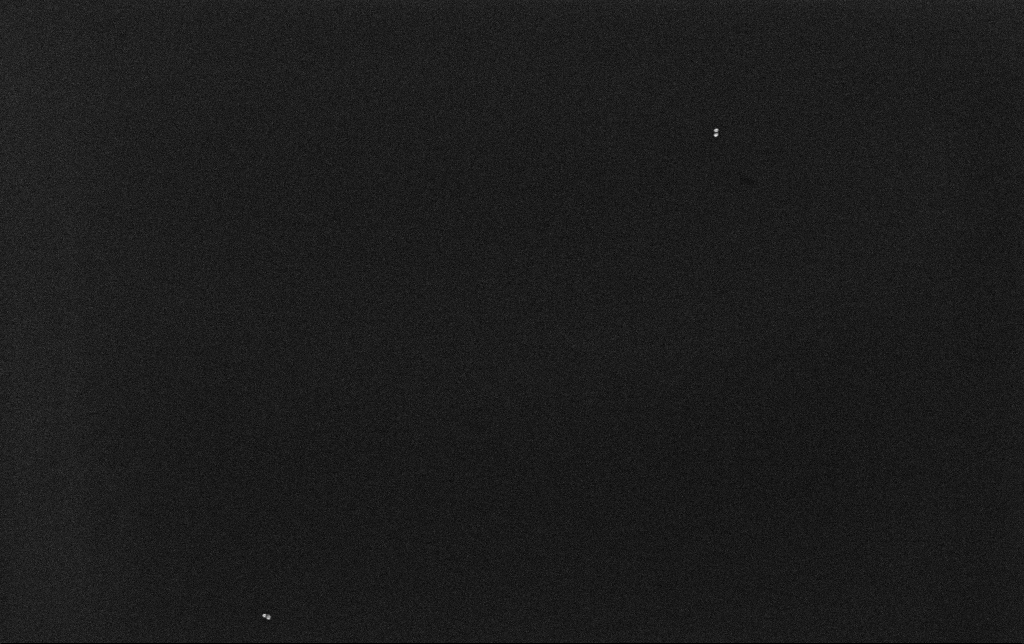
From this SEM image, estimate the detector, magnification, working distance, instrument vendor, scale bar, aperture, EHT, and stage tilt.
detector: InLens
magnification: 100 K X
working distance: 3.2 mm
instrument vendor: Zeiss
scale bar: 200 nm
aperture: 30 µm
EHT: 10 kV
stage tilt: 0°